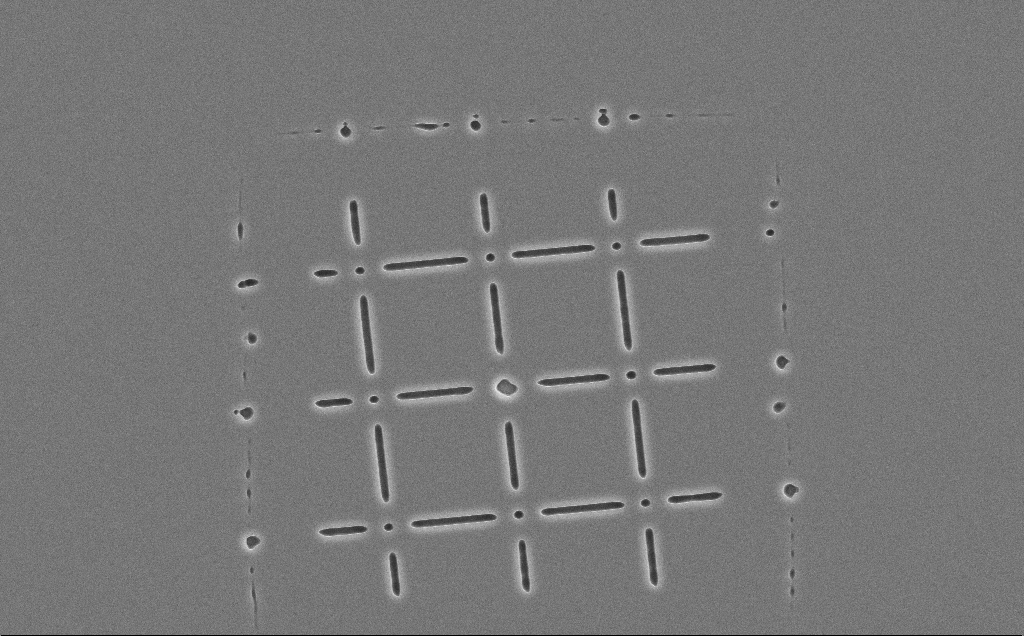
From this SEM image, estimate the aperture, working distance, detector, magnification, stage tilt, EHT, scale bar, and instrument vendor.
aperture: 30 µm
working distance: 15 mm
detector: SE2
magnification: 2.34 K X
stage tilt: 0°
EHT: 10 kV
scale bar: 10000 nm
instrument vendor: Zeiss